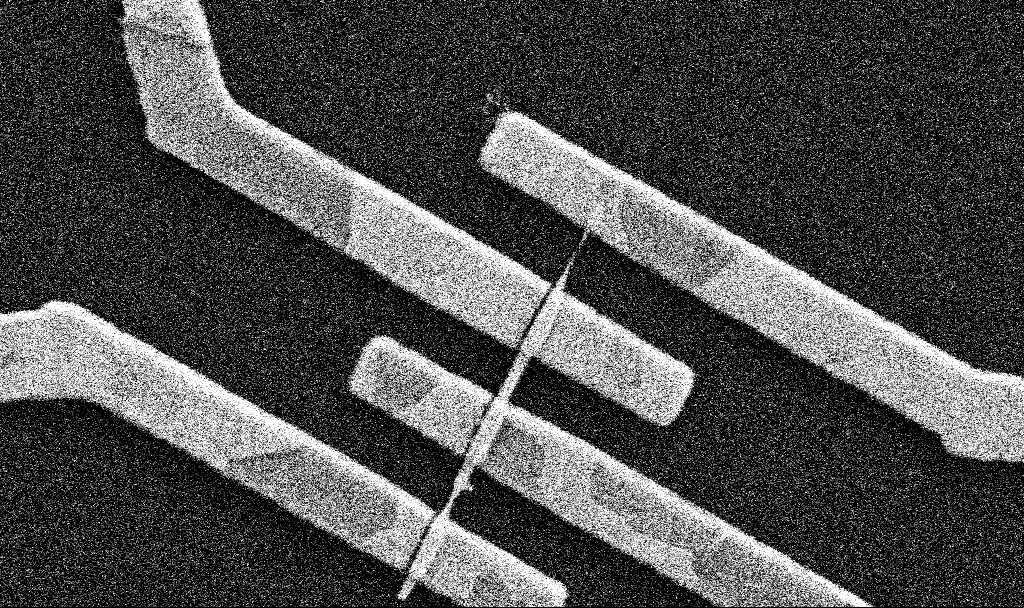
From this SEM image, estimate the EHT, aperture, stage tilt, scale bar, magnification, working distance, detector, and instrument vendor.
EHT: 5 kV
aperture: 30 µm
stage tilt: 0°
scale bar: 1000 nm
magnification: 42.97 K X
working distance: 8.5 mm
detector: SE2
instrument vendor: Zeiss